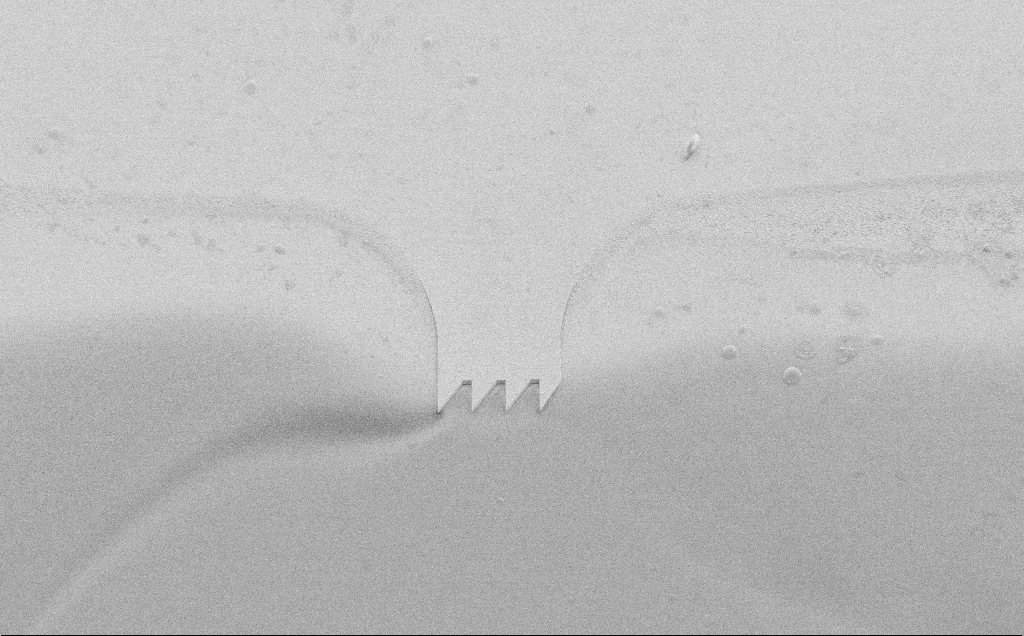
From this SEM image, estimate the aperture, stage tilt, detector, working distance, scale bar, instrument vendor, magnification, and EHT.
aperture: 30 µm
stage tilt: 45°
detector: SE2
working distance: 8 mm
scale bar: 20000 nm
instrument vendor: Zeiss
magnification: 1.08 K X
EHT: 10 kV